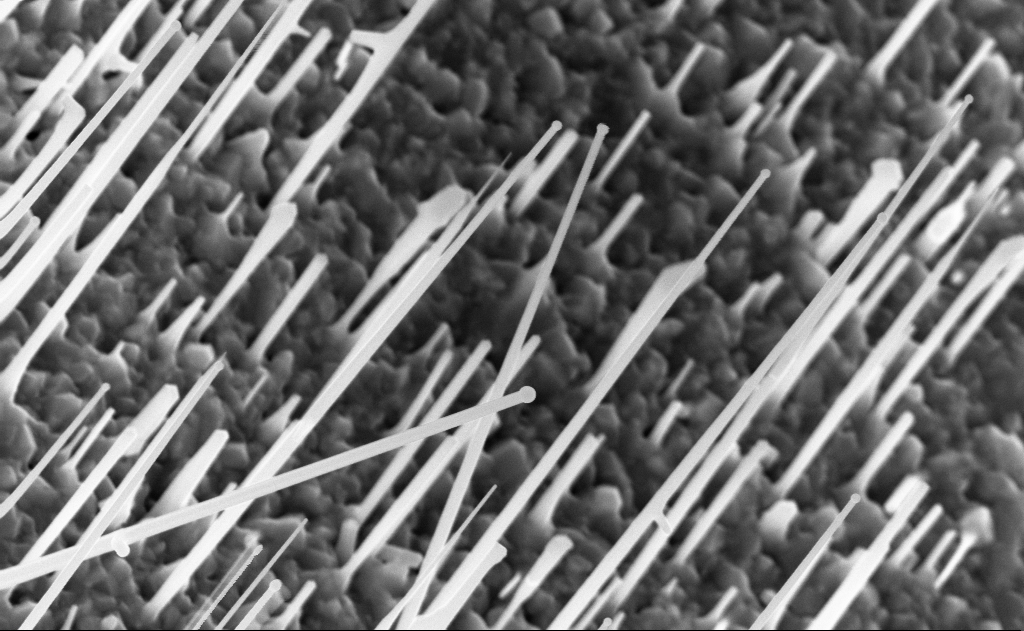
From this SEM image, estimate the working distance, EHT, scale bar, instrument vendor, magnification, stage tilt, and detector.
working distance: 10 mm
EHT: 10 kV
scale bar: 1000 nm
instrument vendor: Zeiss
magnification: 40 K X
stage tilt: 0°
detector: InLens